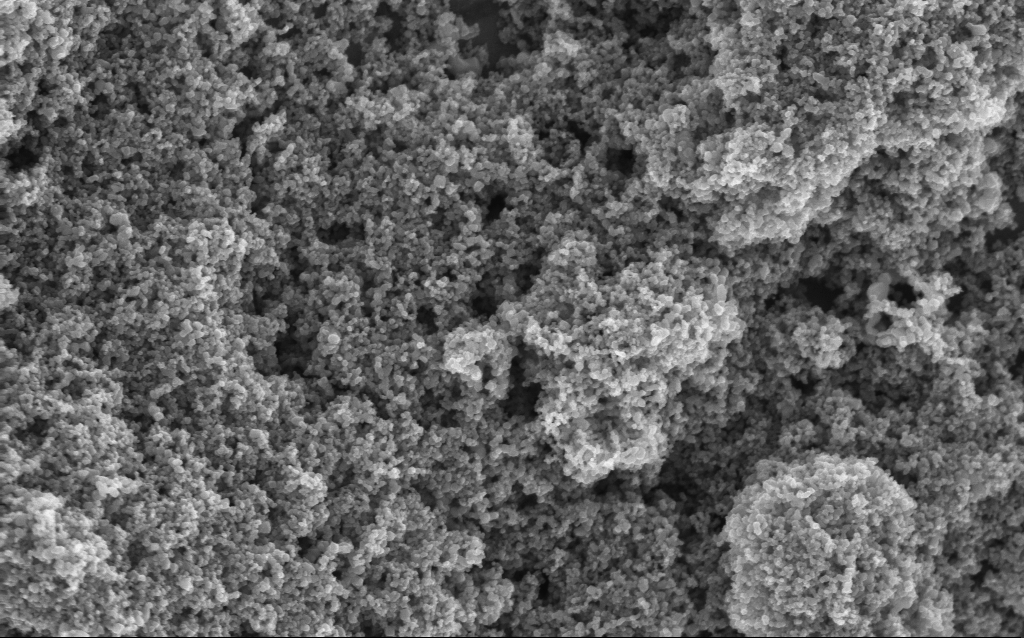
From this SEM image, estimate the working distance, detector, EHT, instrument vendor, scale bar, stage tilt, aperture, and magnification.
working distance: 4.2 mm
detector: InLens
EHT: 5 kV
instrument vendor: Zeiss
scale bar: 1000 nm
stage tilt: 0°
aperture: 30 µm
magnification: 68.59 K X